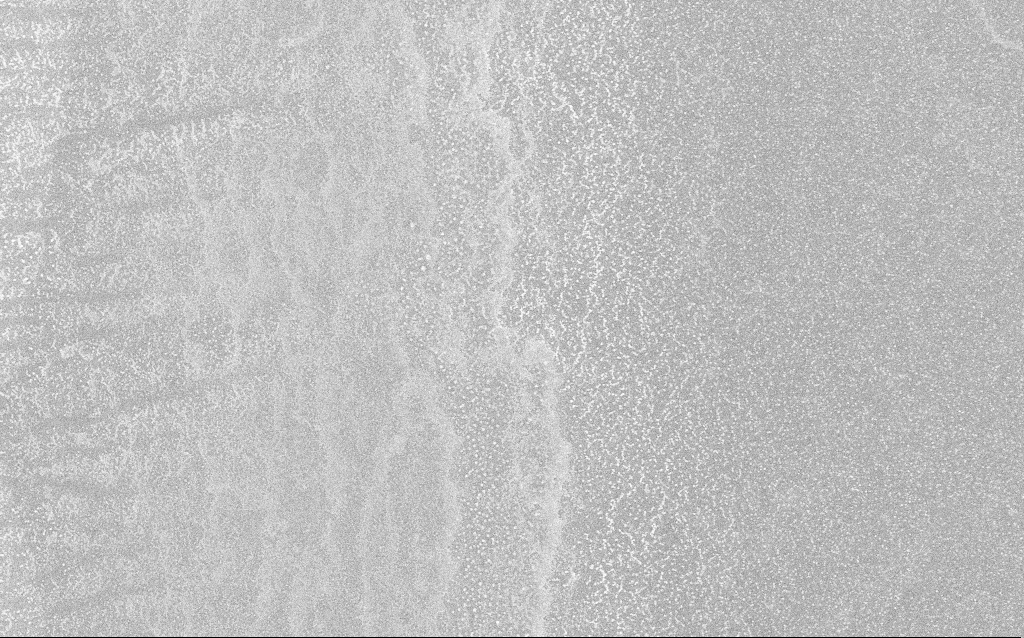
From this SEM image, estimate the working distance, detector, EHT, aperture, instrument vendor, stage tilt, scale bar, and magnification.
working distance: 1.8 mm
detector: InLens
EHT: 20 kV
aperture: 30 µm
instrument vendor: Zeiss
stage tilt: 0°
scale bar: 20000 nm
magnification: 1 K X